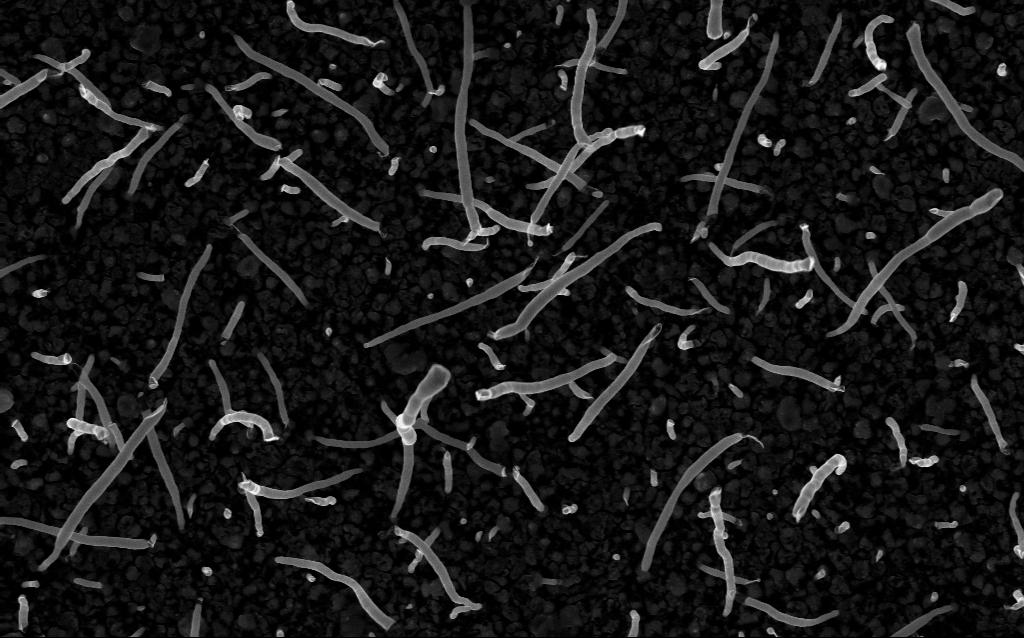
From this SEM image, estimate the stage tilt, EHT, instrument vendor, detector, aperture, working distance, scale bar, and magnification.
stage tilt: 0°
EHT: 5 kV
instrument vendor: Zeiss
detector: InLens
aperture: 30 µm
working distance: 2.2 mm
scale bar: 1000 nm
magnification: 50 K X